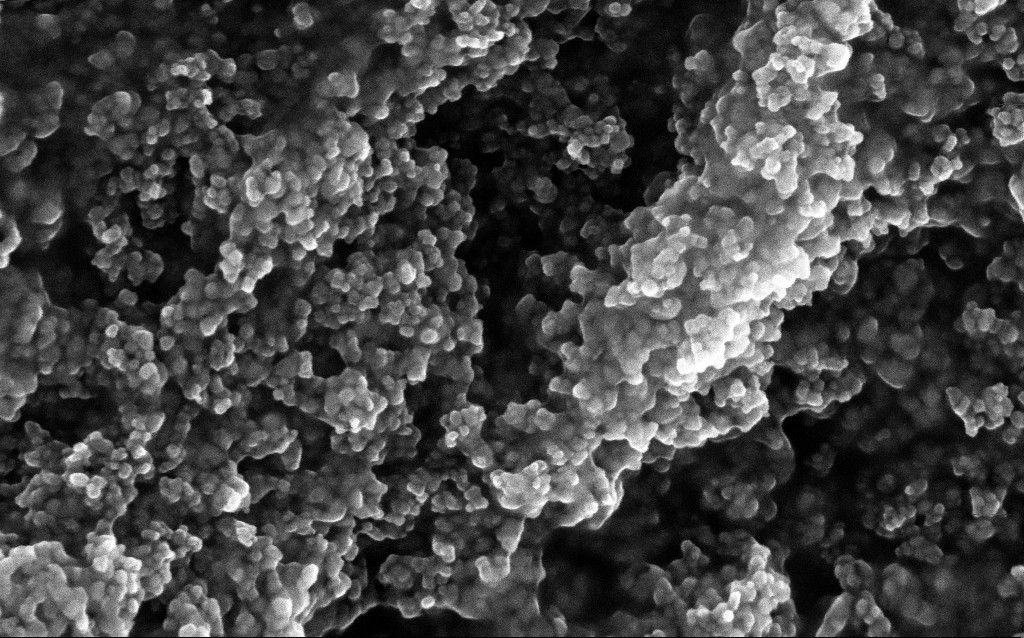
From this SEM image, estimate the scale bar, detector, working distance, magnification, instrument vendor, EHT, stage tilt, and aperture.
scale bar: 100 nm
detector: InLens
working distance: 2.6 mm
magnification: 204.13 K X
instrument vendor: Zeiss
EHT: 10 kV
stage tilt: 0°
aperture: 30 µm